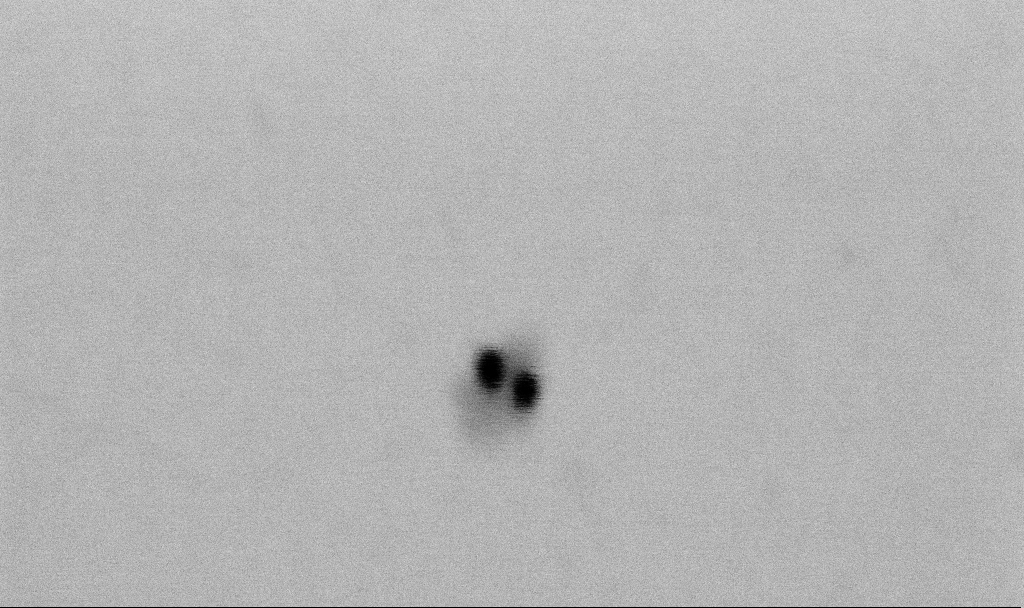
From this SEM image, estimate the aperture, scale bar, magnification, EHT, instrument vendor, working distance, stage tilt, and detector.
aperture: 30 µm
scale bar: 100 nm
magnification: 400 K X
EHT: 2 kV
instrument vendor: Zeiss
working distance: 6.6 mm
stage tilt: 0°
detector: SE2